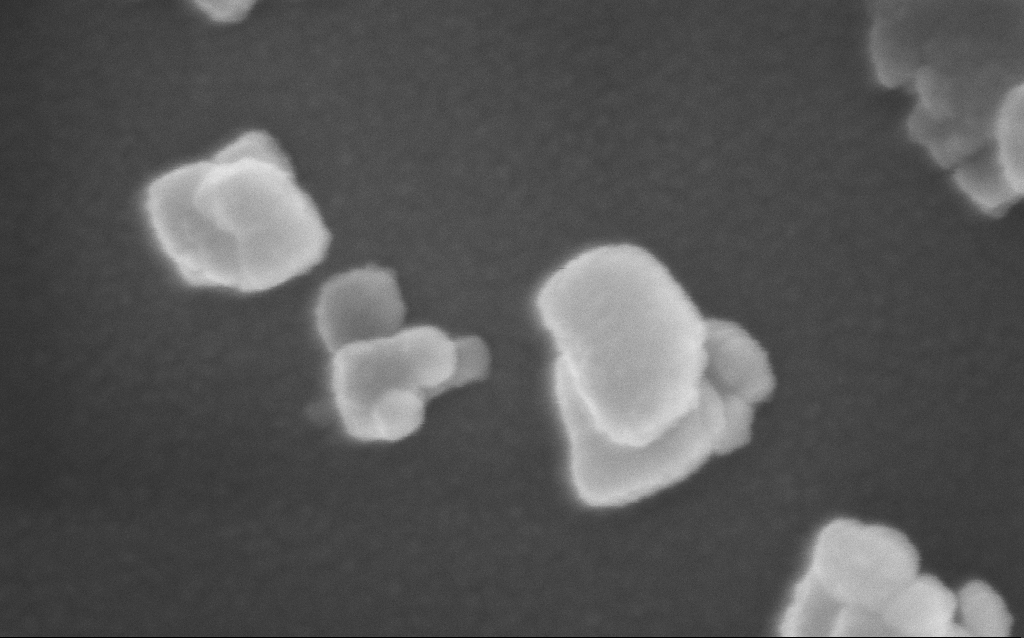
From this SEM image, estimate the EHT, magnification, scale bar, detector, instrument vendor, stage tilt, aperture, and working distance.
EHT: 10 kV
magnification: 316.87 K X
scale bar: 100 nm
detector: InLens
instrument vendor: Zeiss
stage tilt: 0°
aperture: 30 µm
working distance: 6 mm